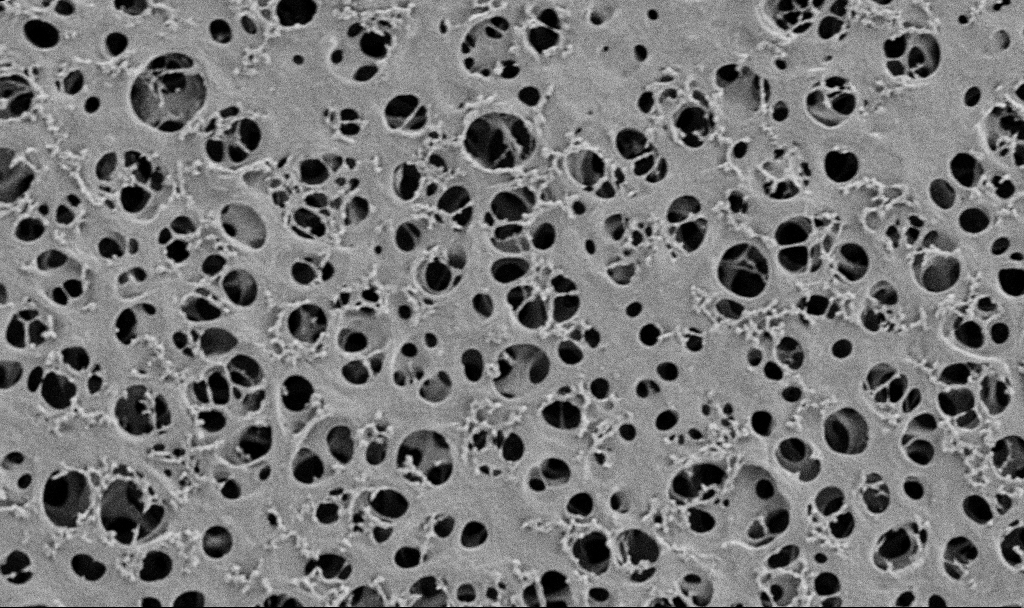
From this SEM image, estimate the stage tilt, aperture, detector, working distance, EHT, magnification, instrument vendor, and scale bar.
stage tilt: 0°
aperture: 30 µm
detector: SE2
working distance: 3.7 mm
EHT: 2 kV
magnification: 10 K X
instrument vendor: Zeiss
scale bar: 2000 nm